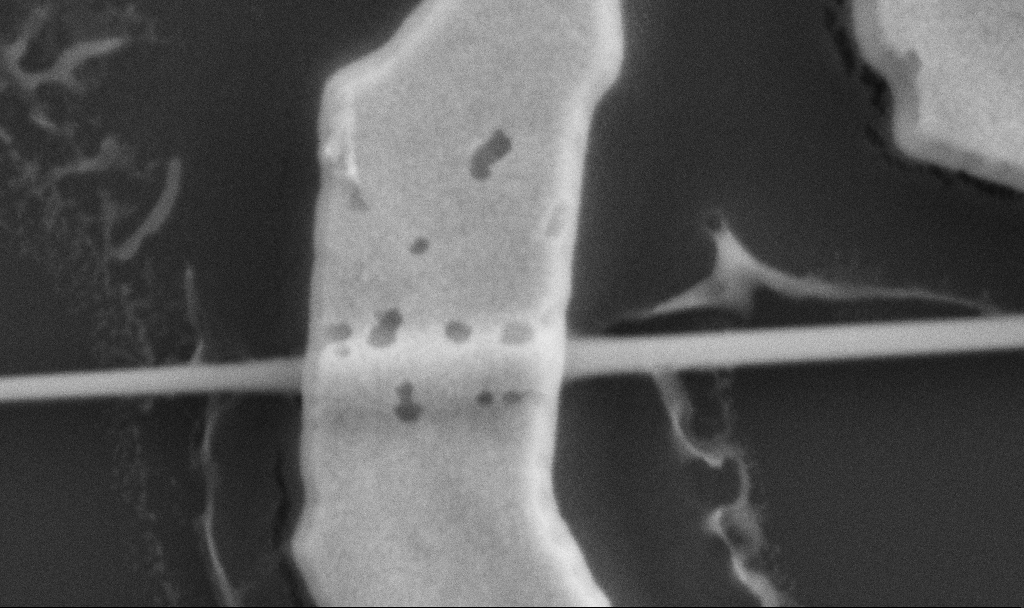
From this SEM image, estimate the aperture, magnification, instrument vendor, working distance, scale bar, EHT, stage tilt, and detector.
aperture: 30 µm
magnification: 125.97 K X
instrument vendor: Zeiss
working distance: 10.7 mm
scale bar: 200 nm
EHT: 5 kV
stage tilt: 0°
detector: SE2